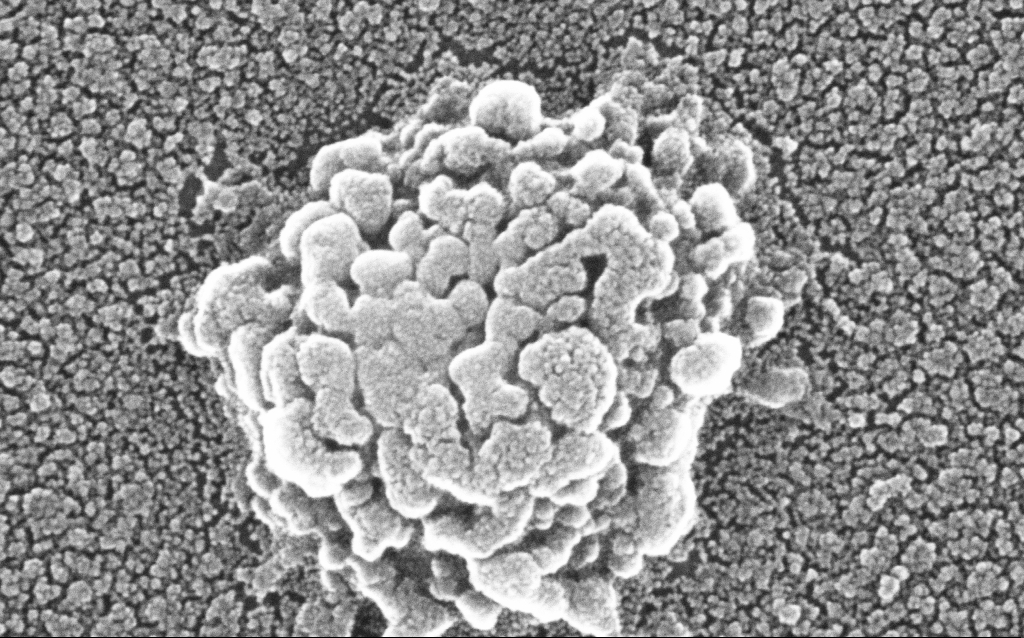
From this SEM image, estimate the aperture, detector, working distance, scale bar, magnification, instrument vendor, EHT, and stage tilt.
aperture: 30 µm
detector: InLens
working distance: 1.8 mm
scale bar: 100 nm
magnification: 500 K X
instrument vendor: Zeiss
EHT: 20 kV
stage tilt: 0°